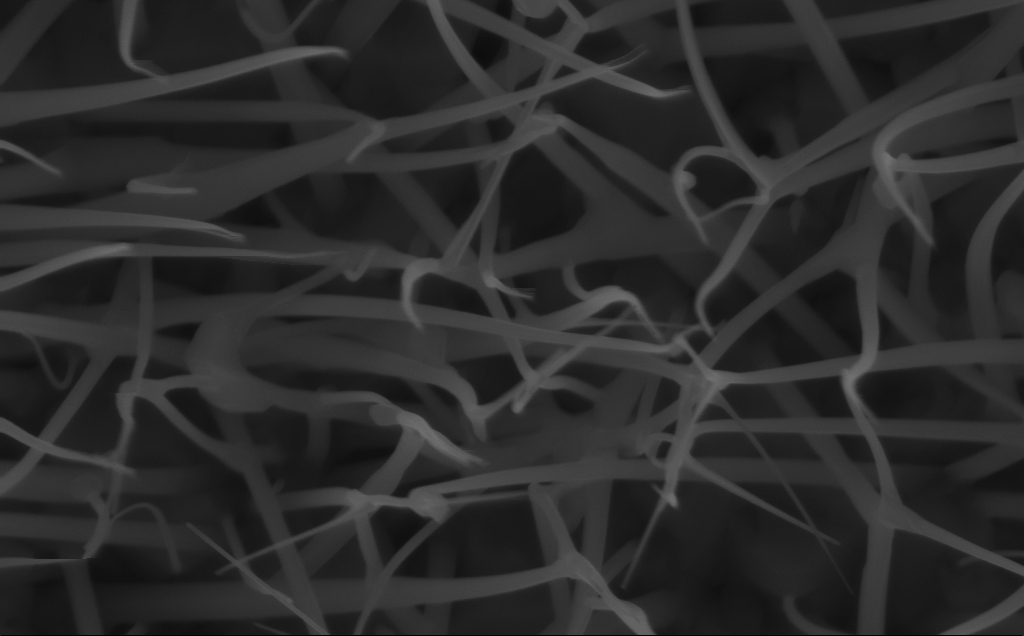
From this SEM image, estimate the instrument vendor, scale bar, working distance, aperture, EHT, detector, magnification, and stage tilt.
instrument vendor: Zeiss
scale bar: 200 nm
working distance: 6 mm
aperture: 30 µm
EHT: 10 kV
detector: InLens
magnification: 80 K X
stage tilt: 0°